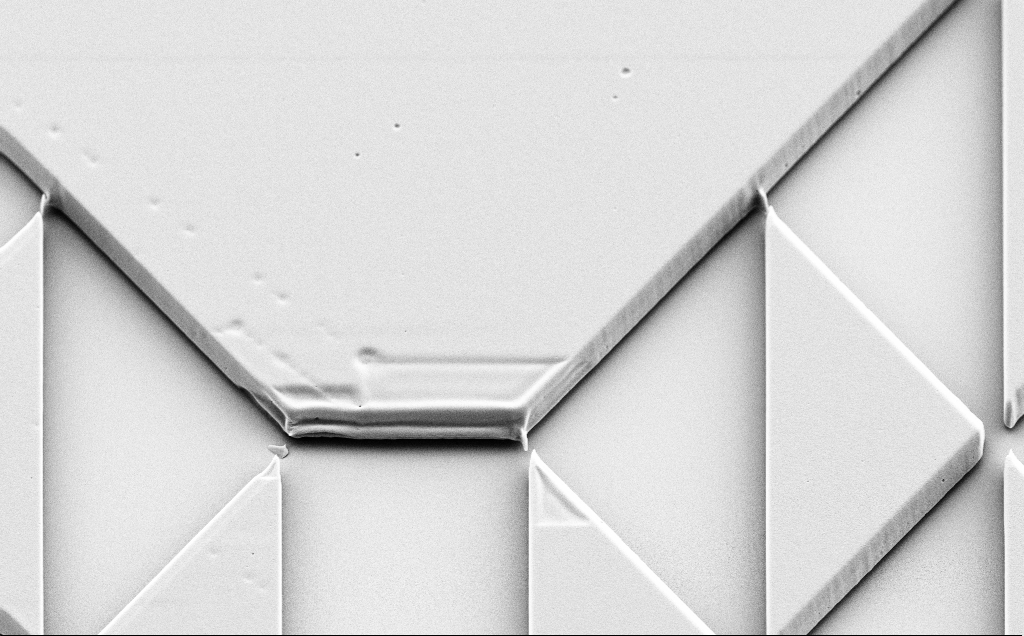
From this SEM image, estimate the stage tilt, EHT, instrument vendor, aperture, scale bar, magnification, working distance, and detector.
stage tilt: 40°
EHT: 5 kV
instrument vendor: Zeiss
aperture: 30 µm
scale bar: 10000 nm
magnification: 2.48 K X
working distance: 9 mm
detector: SE2